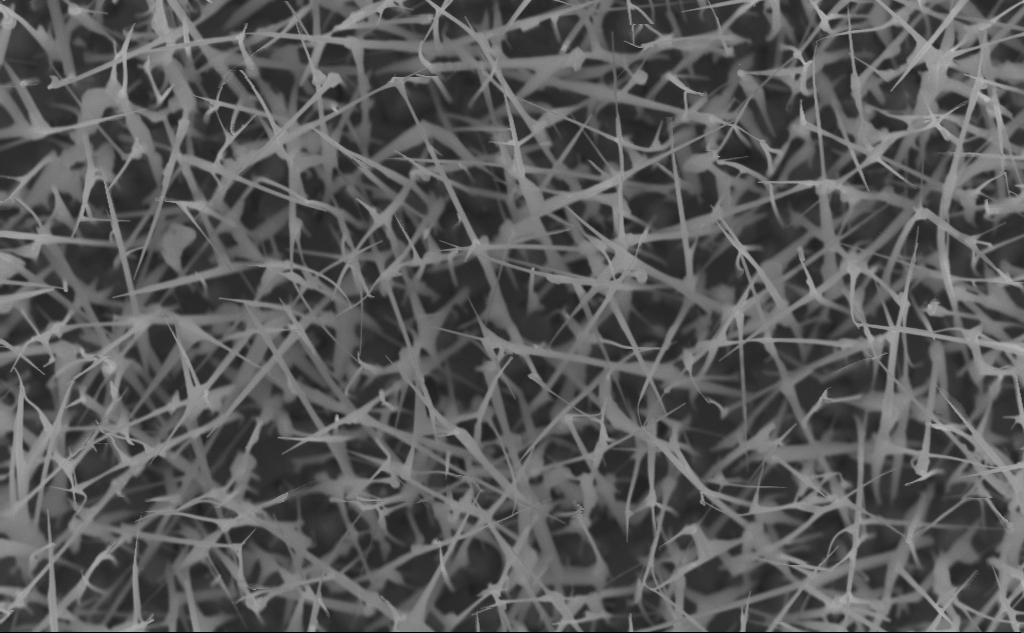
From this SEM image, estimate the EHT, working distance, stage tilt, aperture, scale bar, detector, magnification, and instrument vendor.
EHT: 10 kV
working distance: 6 mm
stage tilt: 0°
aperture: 30 µm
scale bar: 1000 nm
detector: InLens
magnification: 40 K X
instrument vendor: Zeiss